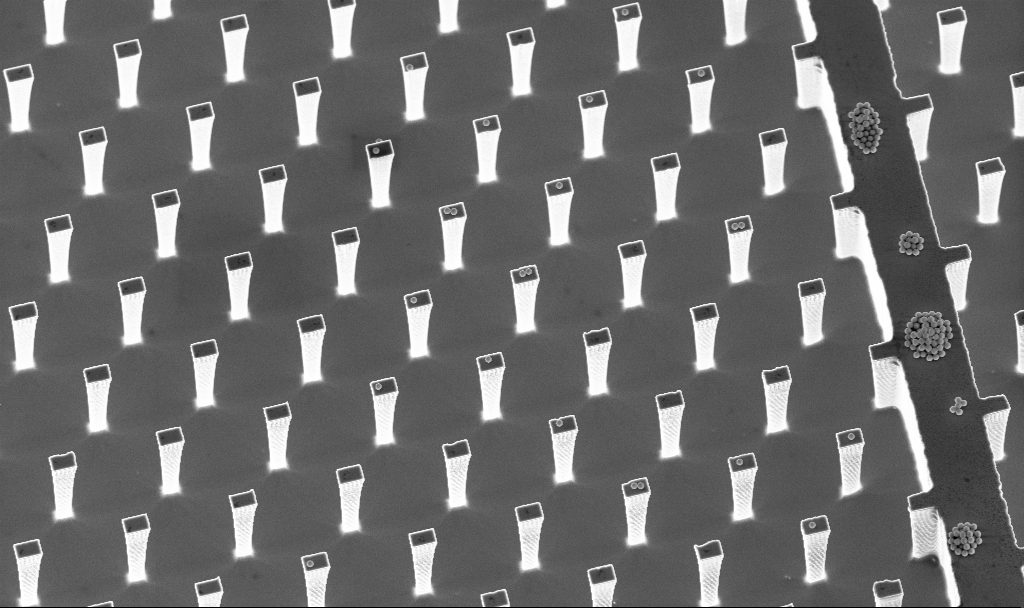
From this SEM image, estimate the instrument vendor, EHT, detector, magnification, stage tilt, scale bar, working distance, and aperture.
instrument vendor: Zeiss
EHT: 5 kV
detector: InLens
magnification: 3.37 K X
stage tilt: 20°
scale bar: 20000 nm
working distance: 4.7 mm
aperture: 30 µm